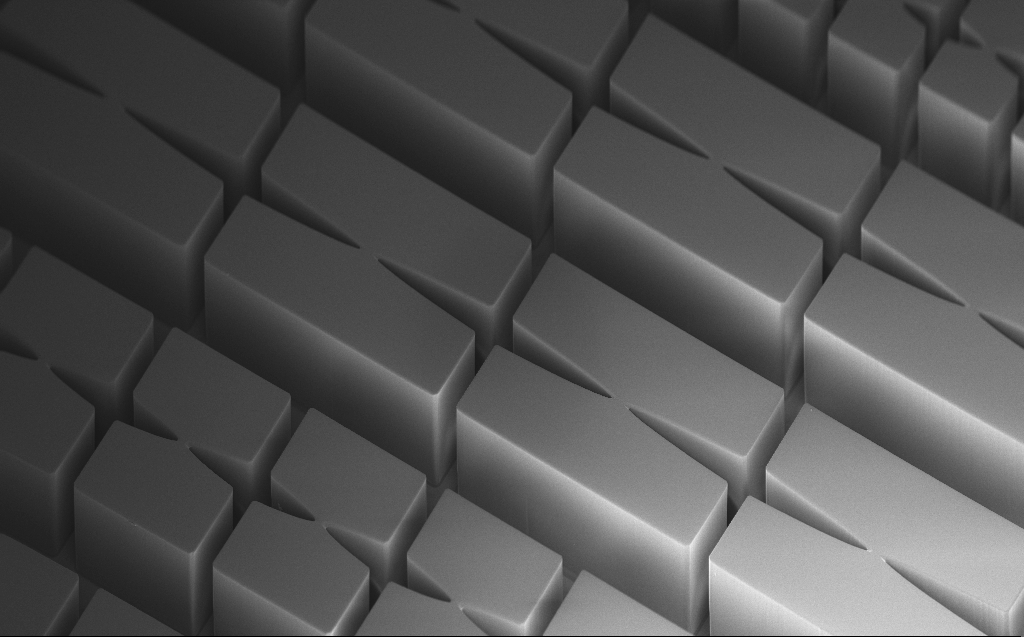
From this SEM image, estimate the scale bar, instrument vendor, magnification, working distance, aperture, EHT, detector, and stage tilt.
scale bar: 100000 nm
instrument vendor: Zeiss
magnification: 0.183 K X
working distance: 6 mm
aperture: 30 µm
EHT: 3 kV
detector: InLens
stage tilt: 45°